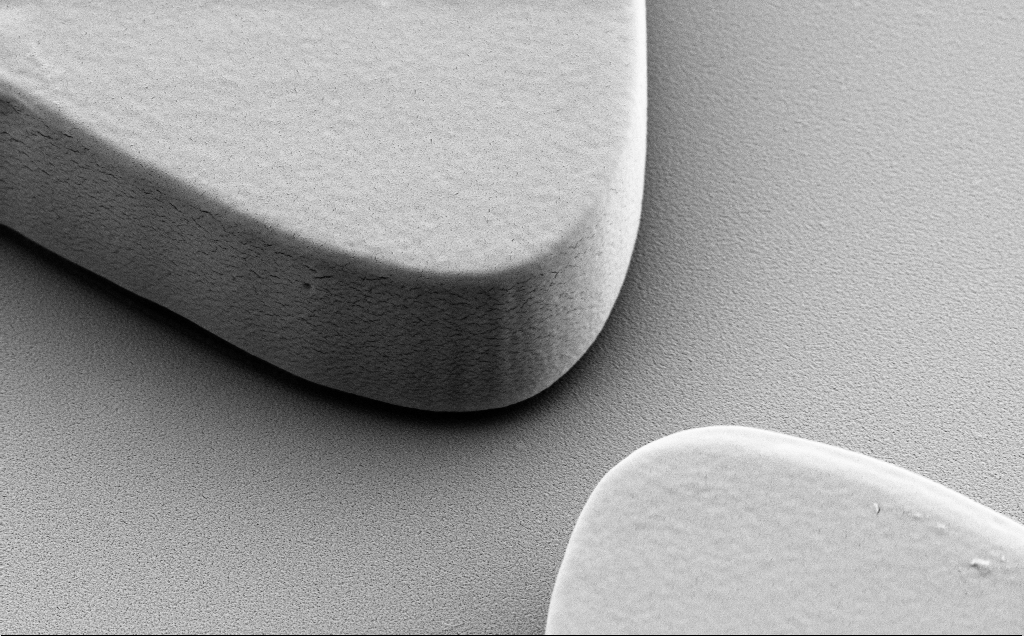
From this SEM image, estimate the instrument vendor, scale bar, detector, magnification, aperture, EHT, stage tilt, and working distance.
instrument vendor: Zeiss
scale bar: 1000 nm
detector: SE2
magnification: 15 K X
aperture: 30 µm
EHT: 5 kV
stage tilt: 40°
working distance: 9 mm